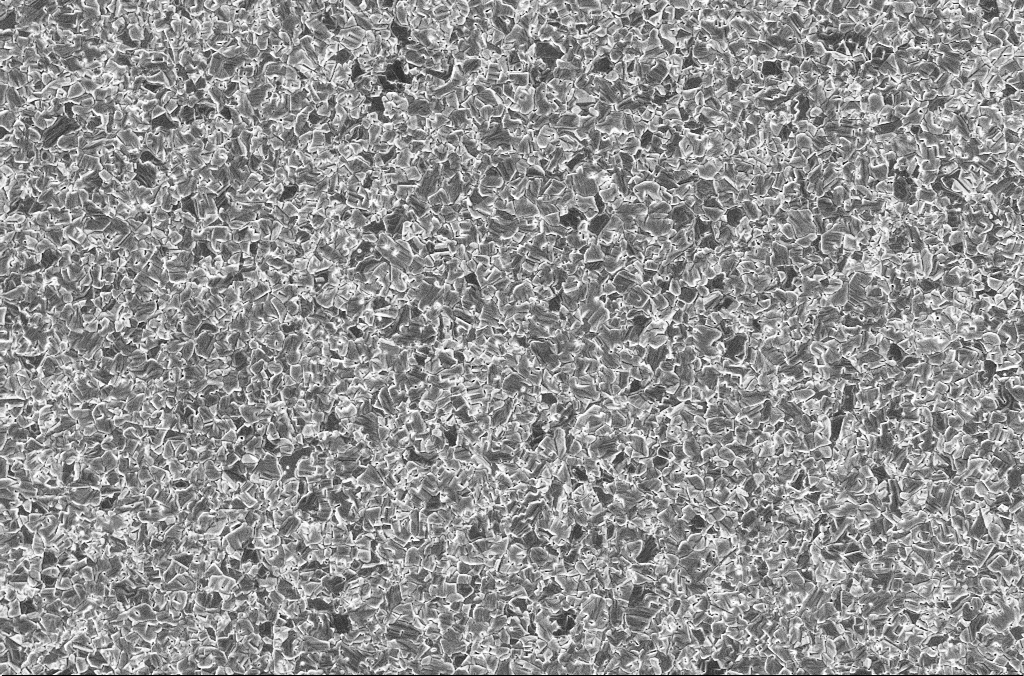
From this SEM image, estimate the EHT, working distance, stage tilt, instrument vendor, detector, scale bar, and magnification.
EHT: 2 kV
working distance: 3 mm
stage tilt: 0°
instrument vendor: Zeiss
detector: InLens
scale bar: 2000 nm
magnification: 10 K X